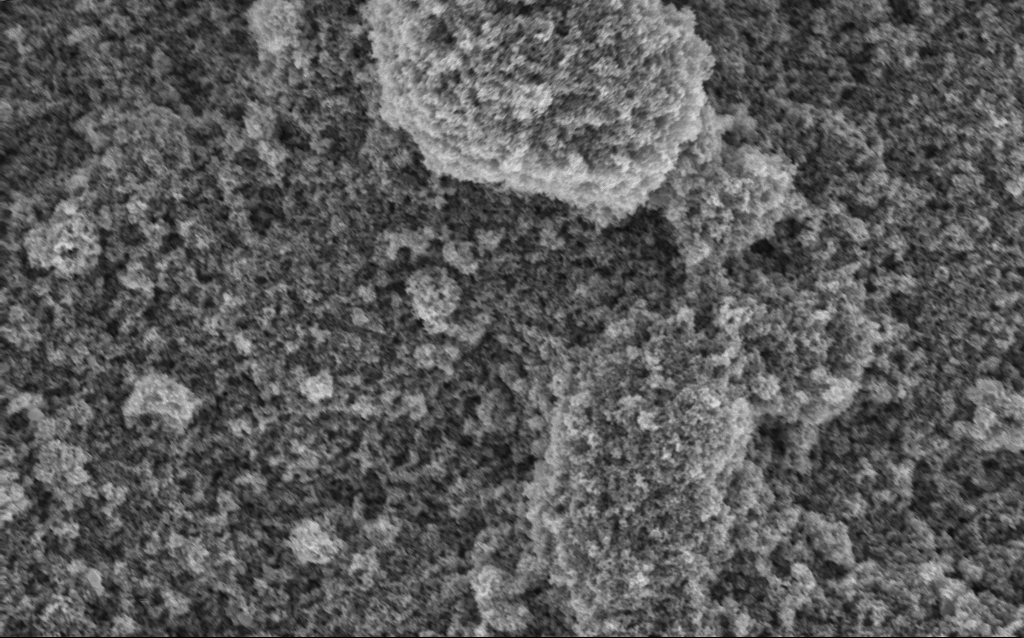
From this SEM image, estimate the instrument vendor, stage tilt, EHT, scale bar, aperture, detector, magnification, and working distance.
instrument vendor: Zeiss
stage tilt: -0°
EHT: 5 kV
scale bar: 2000 nm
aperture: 30 µm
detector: InLens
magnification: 31.56 K X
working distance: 4.2 mm